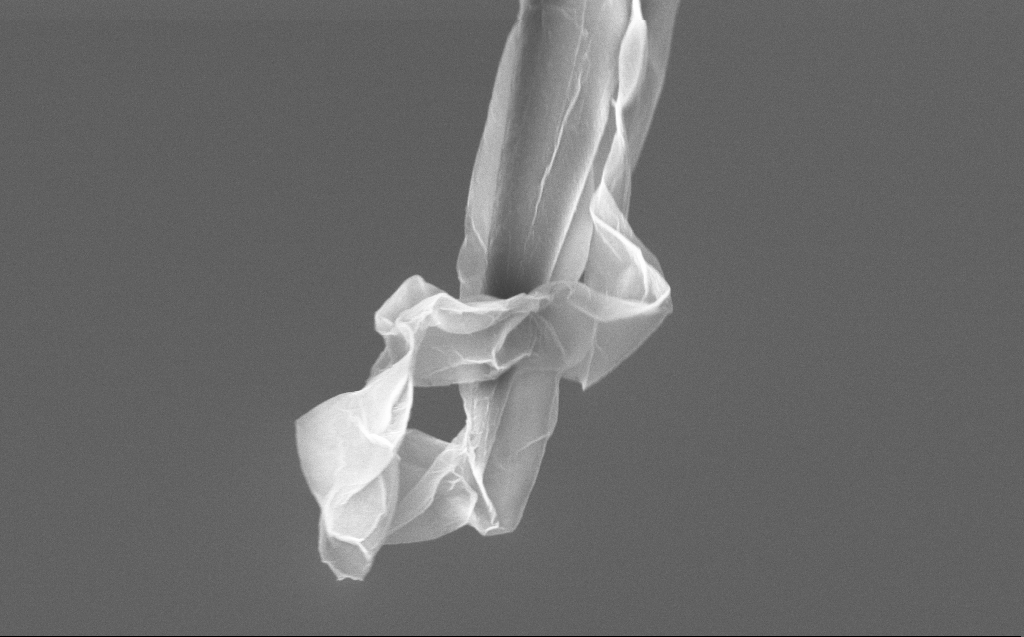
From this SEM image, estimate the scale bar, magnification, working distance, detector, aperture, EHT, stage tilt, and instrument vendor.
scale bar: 1000 nm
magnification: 34.03 K X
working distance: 6 mm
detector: InLens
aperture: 30 µm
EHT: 5 kV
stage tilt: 45°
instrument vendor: Zeiss